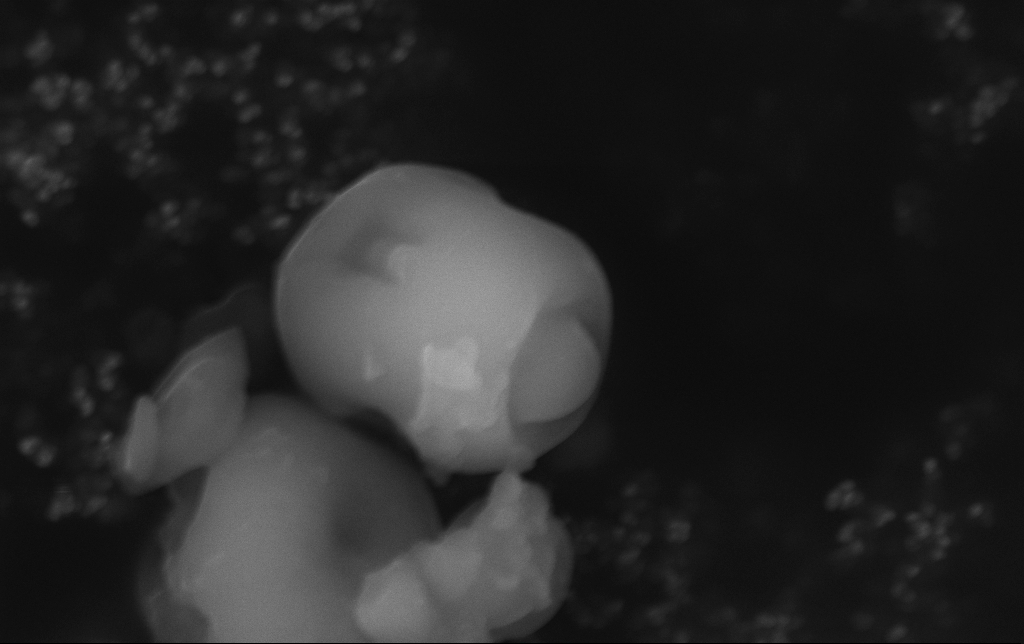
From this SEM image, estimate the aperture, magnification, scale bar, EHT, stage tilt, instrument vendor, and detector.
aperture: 30 µm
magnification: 41.5 K X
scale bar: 1000 nm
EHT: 15 kV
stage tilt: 0°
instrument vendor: Zeiss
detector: InLens